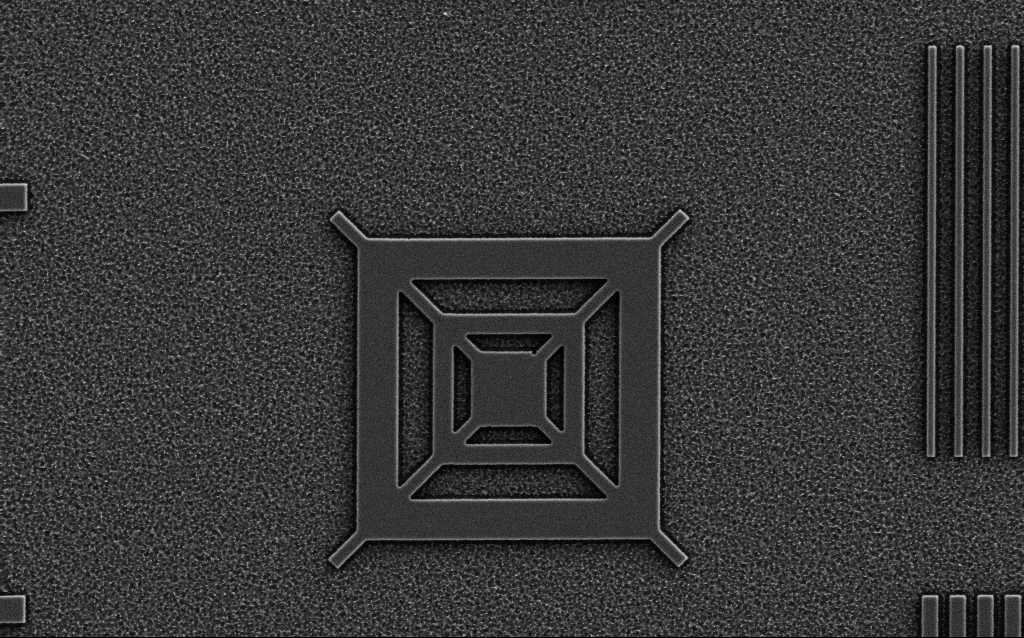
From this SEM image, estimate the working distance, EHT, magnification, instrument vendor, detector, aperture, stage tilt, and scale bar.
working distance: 5 mm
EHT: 3 kV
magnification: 10.17 K X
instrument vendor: Zeiss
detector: SE2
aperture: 30 µm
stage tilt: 0°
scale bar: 2000 nm